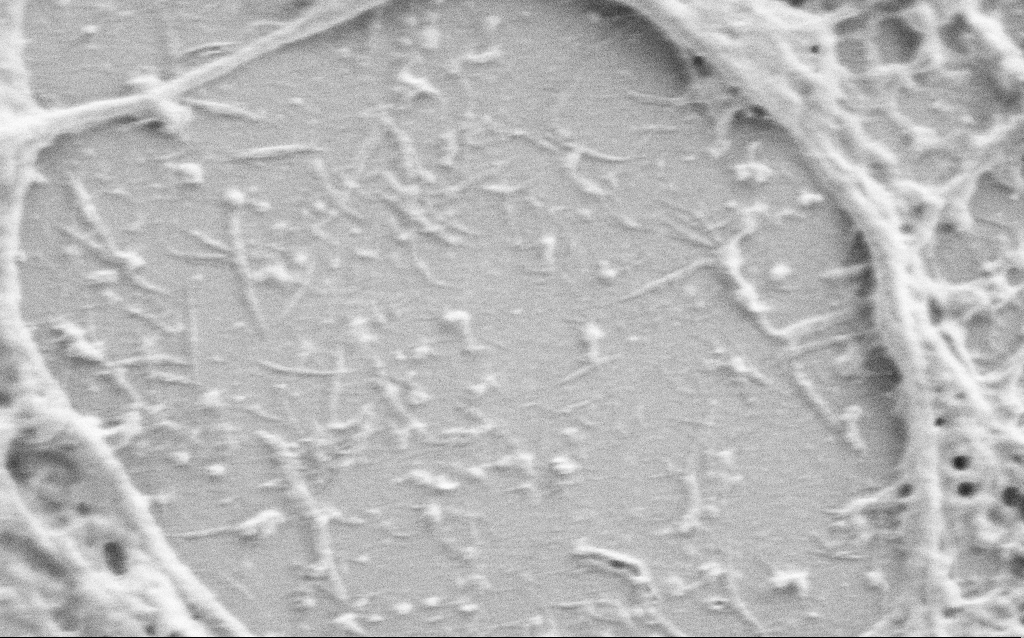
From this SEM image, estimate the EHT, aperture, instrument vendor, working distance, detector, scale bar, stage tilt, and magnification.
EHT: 0.8 kV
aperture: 30 µm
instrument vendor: Zeiss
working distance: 5.7 mm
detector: SE2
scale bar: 1000 nm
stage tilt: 0°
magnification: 40 K X